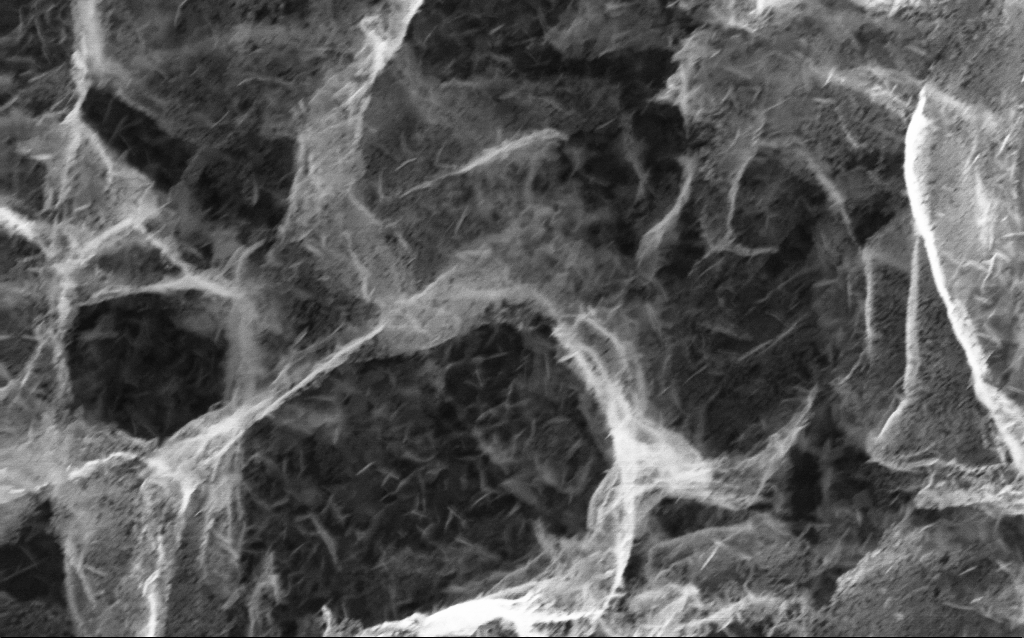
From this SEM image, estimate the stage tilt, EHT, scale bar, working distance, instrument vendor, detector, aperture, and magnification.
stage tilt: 0°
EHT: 10 kV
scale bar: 200 nm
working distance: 2.8 mm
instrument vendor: Zeiss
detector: InLens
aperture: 30 µm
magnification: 80 K X